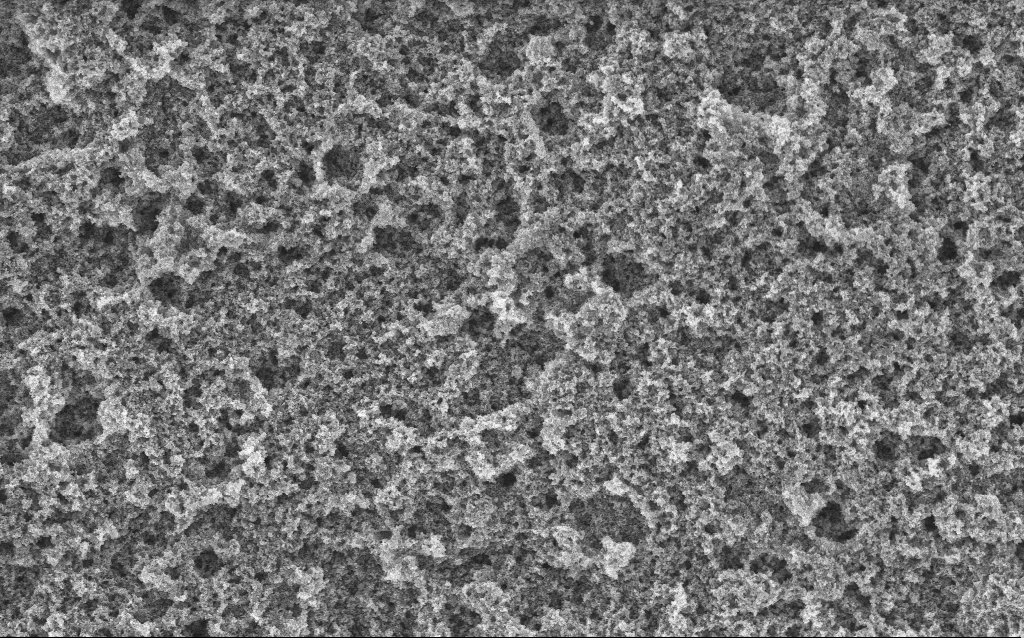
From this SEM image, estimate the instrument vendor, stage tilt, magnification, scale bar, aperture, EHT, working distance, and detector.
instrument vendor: Zeiss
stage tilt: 0°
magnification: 20.87 K X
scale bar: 1000 nm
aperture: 30 µm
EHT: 5 kV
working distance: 4.4 mm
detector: InLens